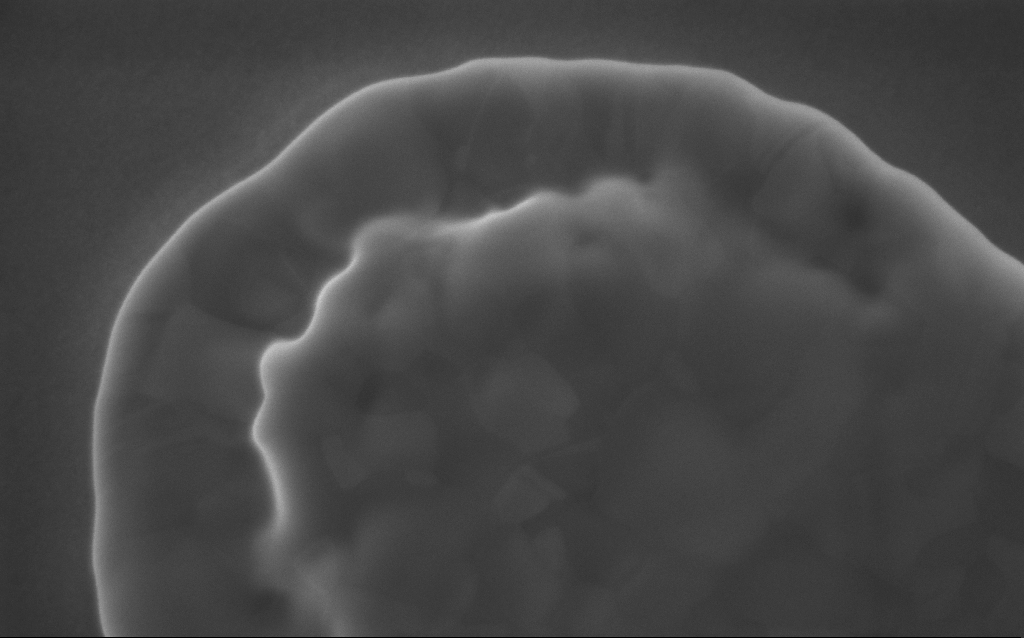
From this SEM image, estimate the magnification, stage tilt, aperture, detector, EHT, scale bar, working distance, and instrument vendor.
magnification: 217 K X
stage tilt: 0°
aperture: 30 µm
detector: InLens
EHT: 5 kV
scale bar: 200 nm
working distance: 3 mm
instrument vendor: Zeiss